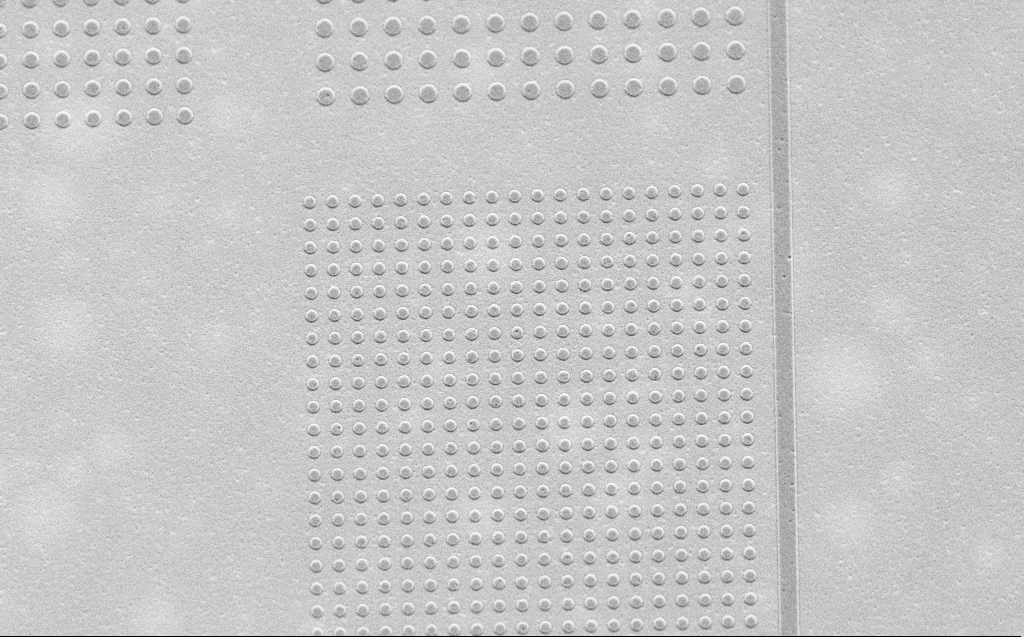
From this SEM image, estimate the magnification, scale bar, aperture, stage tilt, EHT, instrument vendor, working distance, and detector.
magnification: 7.07 K X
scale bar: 10000 nm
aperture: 30 µm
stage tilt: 30°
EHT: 2.5 kV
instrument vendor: Zeiss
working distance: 4 mm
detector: SE2